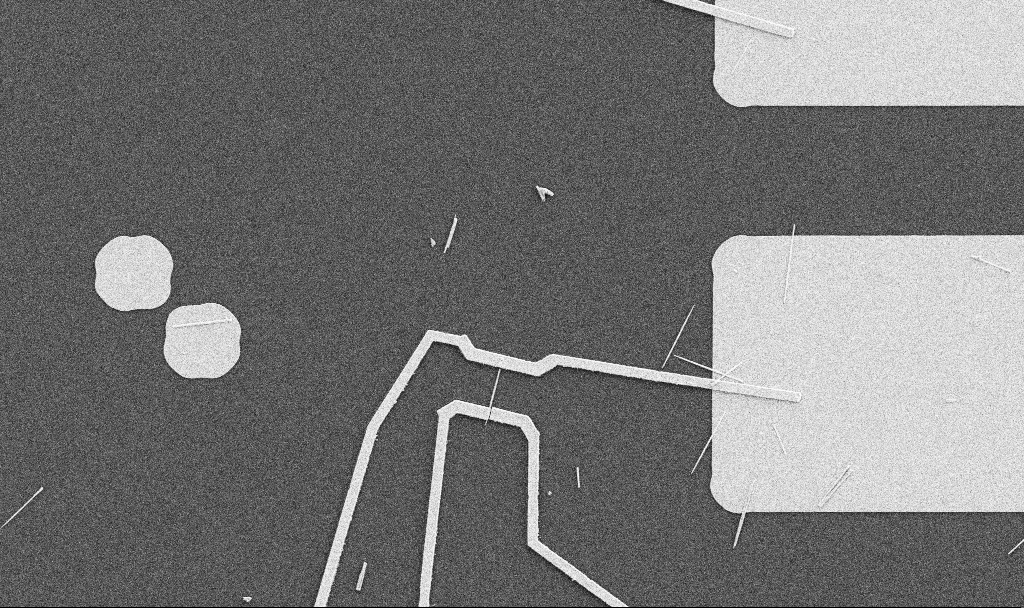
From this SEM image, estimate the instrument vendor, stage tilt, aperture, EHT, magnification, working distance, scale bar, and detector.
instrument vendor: Zeiss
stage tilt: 0°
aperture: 30 µm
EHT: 5 kV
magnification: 5 K X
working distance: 10.7 mm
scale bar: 10000 nm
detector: SE2